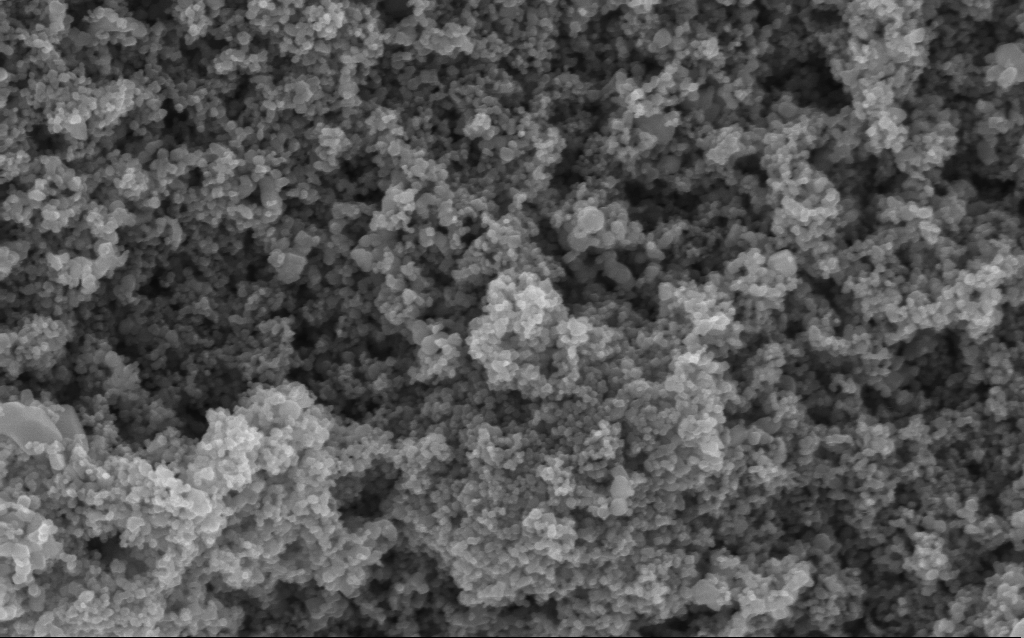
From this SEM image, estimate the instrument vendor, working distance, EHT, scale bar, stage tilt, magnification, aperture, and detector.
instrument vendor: Zeiss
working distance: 4.2 mm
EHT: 5 kV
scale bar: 200 nm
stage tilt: -0°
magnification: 114.56 K X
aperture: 30 µm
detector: InLens